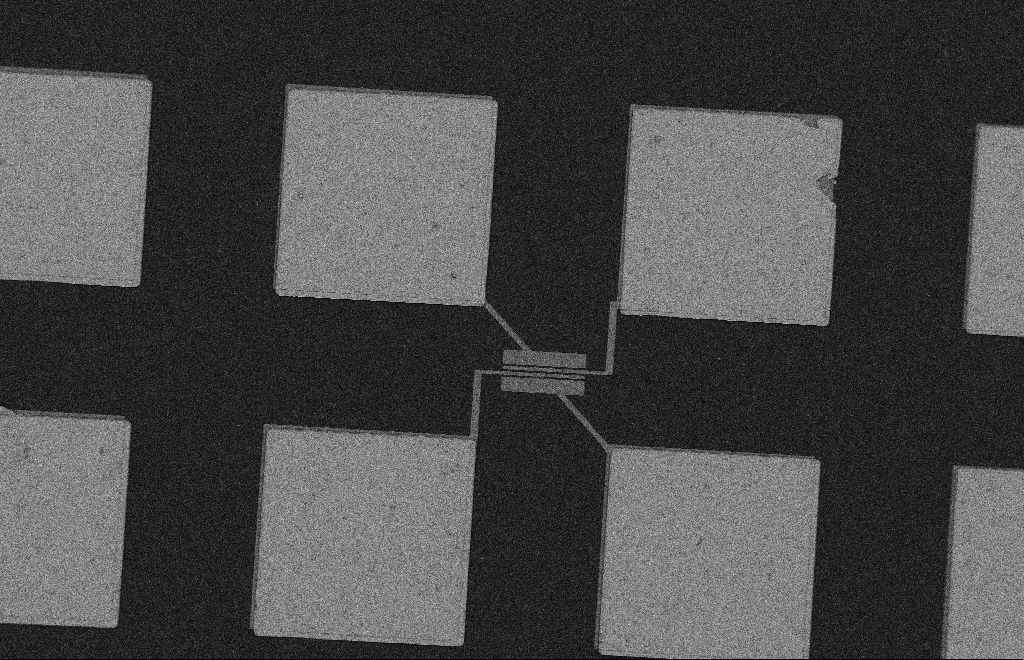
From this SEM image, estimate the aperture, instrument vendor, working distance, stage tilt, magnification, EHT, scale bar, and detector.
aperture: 20 µm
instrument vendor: Zeiss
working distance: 10 mm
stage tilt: -0.3°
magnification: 0.497 K X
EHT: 2 kV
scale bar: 20000 nm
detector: SE2